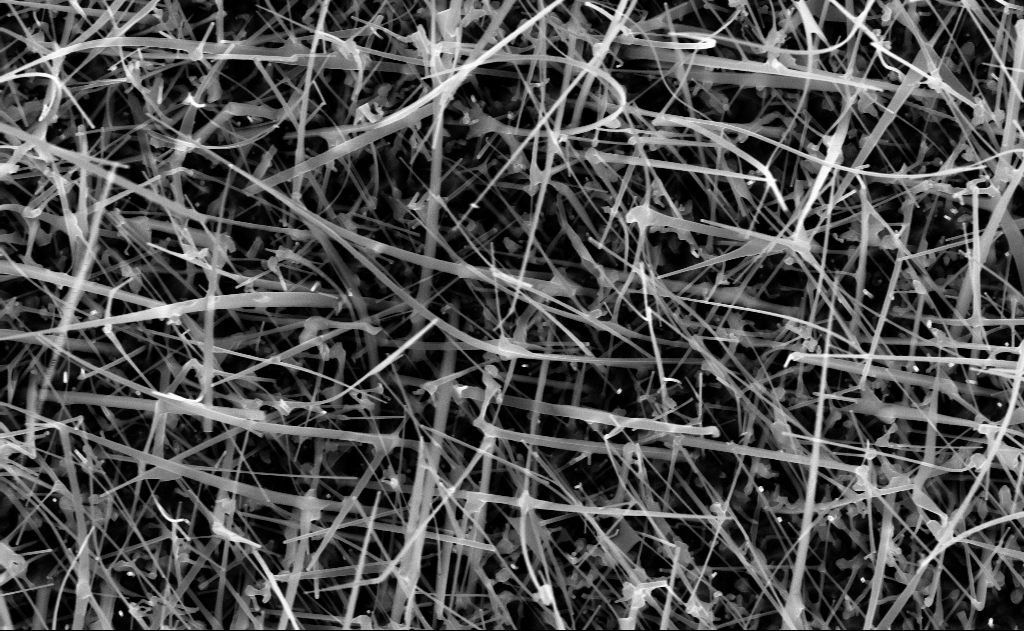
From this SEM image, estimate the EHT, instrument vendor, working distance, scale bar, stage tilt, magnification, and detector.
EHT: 10 kV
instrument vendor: Zeiss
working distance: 9 mm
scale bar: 1000 nm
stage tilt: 0°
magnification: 40 K X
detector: InLens